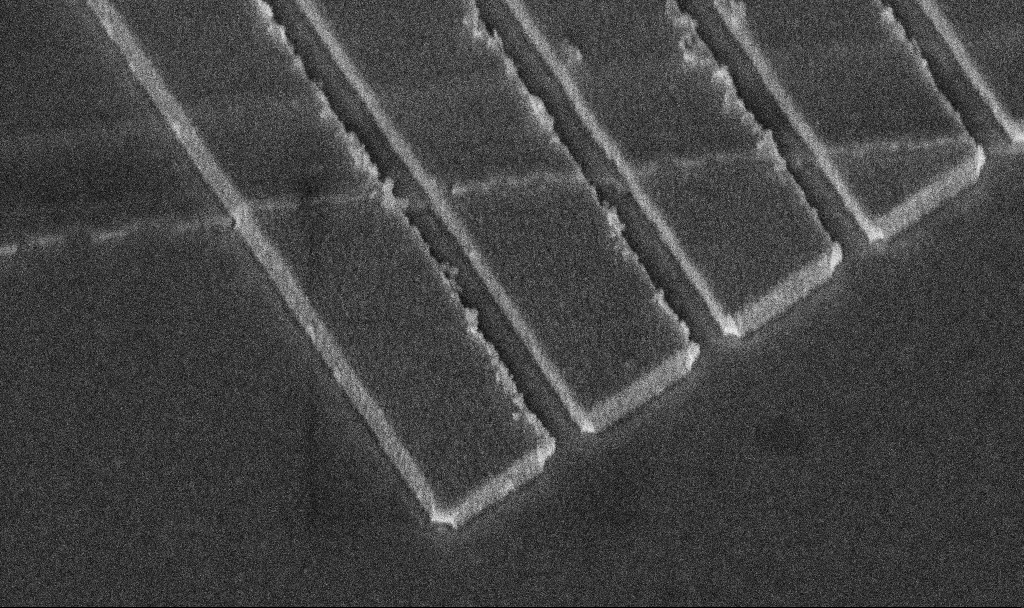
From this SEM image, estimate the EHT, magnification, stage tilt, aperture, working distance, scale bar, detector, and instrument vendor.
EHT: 5 kV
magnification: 77.98 K X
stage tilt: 45°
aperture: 30 µm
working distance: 10.8 mm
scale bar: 200 nm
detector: InLens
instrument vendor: Zeiss